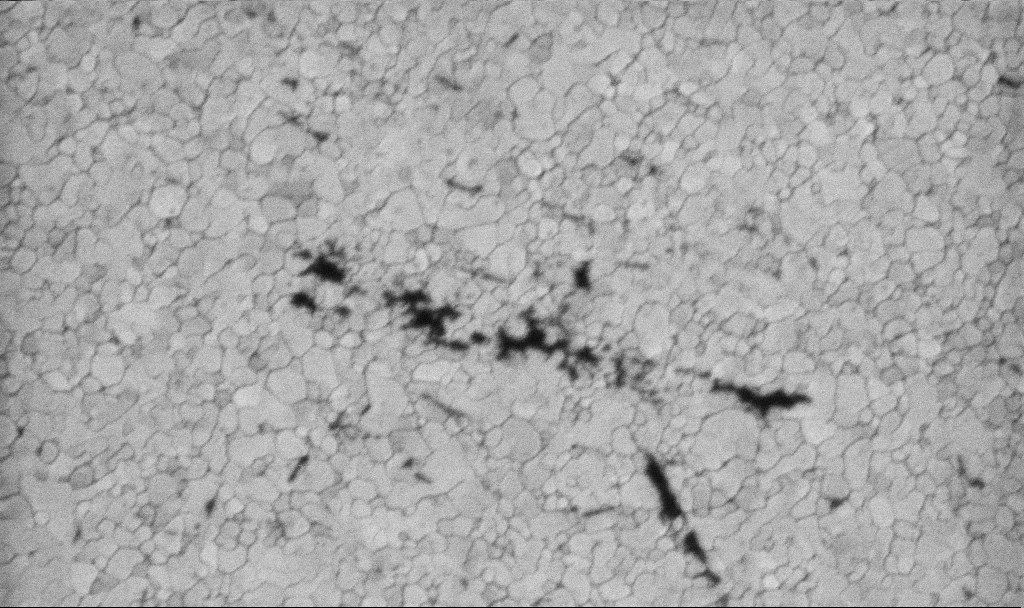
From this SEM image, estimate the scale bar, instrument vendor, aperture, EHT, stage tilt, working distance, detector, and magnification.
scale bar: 200 nm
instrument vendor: Zeiss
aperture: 30 µm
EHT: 5 kV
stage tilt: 0°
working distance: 3.1 mm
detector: InLens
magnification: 100.15 K X